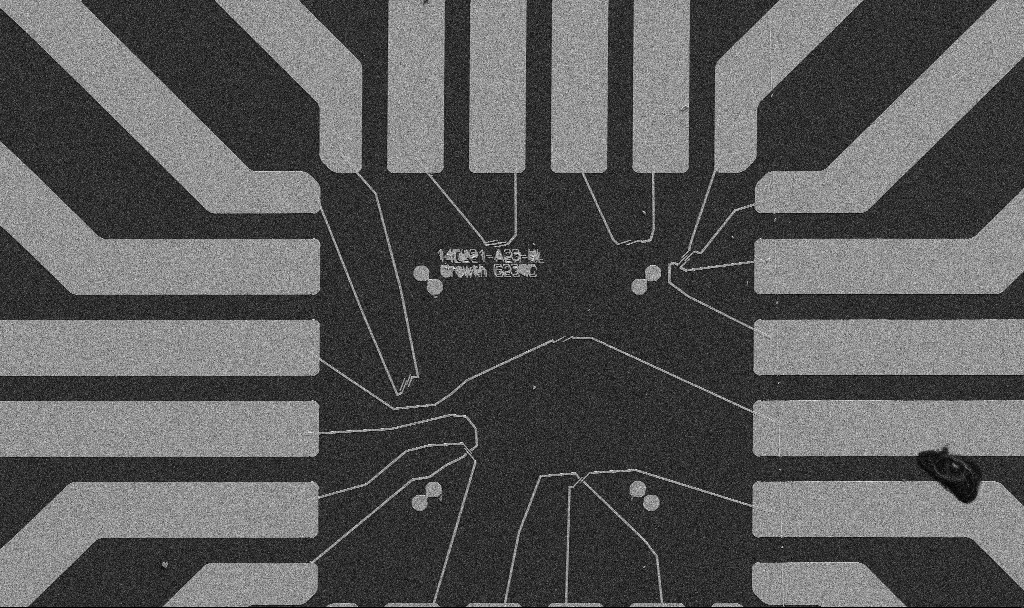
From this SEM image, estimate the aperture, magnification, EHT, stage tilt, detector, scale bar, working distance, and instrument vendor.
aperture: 30 µm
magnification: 1 K X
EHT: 5 kV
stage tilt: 0°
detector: SE2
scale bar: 20000 nm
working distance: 10.7 mm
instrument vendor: Zeiss